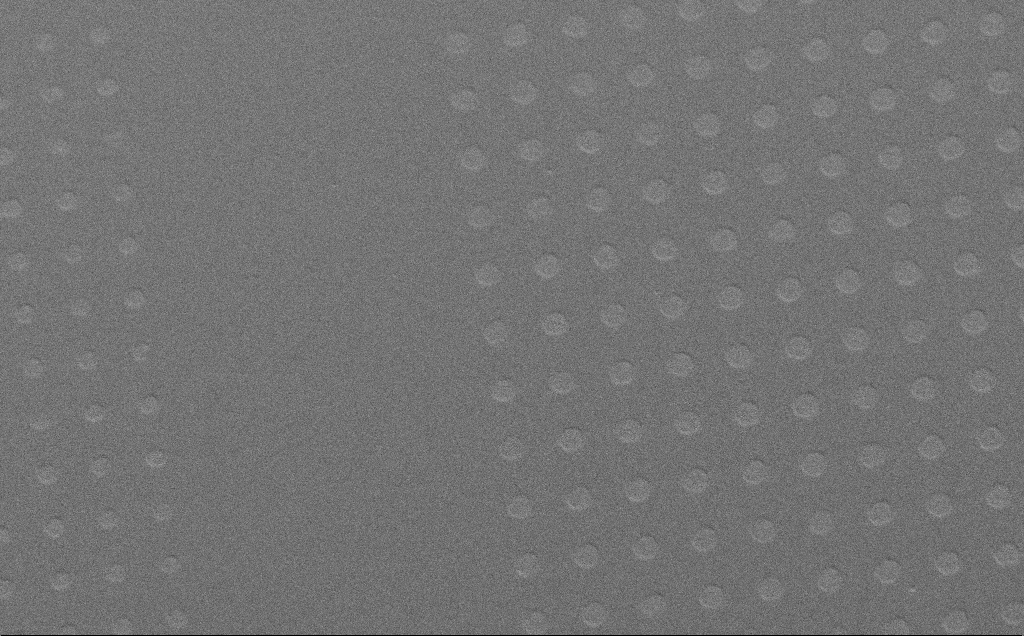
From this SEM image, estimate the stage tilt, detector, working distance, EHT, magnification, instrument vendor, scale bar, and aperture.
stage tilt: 40.4°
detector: SE2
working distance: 6 mm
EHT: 10 kV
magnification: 0.916 K X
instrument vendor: Zeiss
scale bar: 20000 nm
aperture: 30 µm